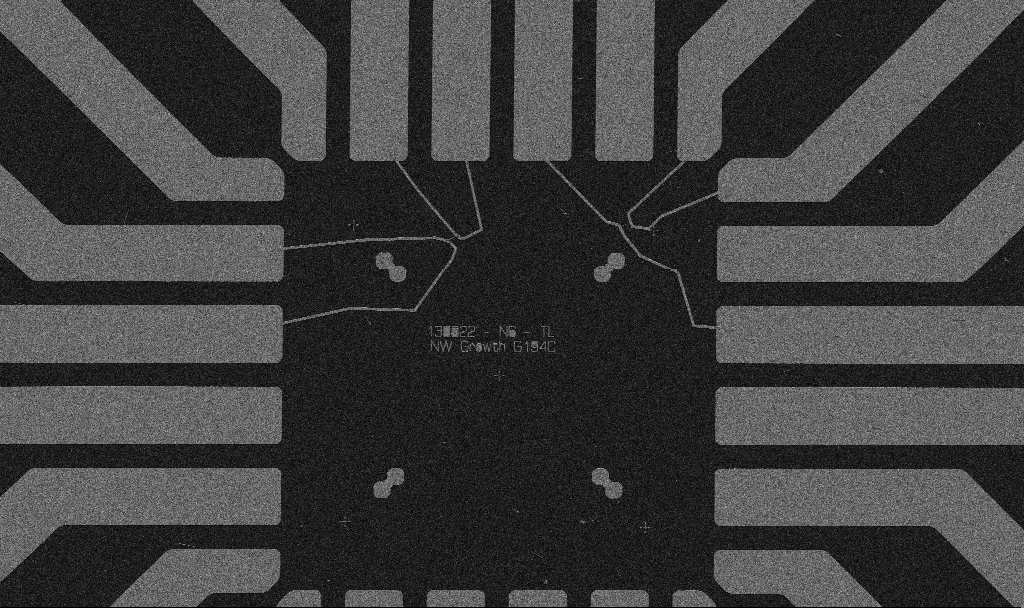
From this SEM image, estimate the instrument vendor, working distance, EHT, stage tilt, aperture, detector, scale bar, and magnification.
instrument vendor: Zeiss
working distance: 10.7 mm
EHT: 5 kV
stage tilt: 0°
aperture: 30 µm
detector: SE2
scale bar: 20000 nm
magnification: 1 K X